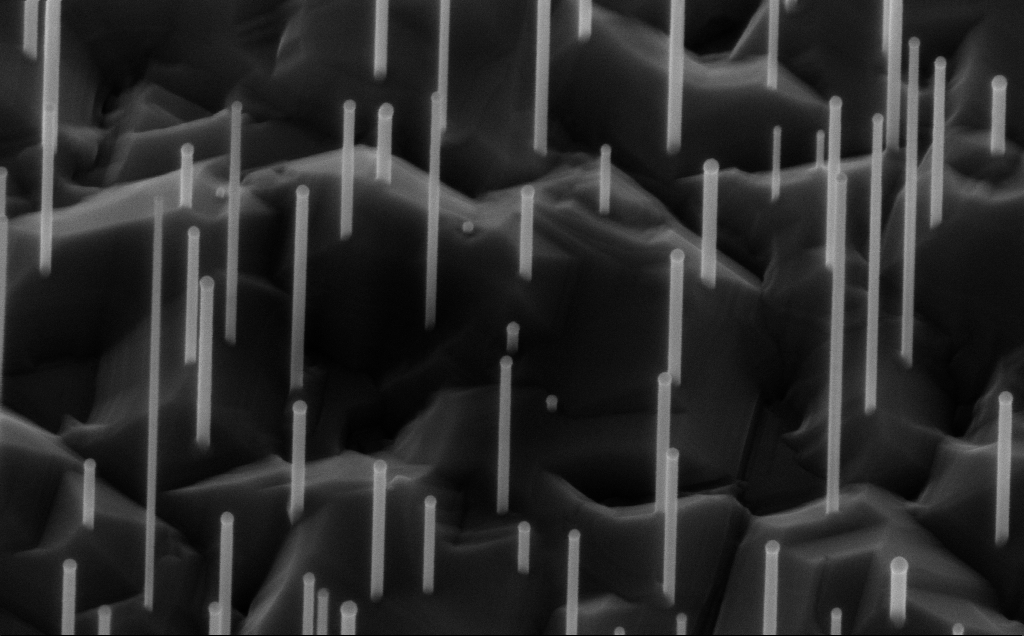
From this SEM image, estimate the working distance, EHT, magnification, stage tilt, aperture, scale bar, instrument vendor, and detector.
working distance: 6 mm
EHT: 10 kV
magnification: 80 K X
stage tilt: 45°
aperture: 30 µm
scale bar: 200 nm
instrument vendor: Zeiss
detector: InLens